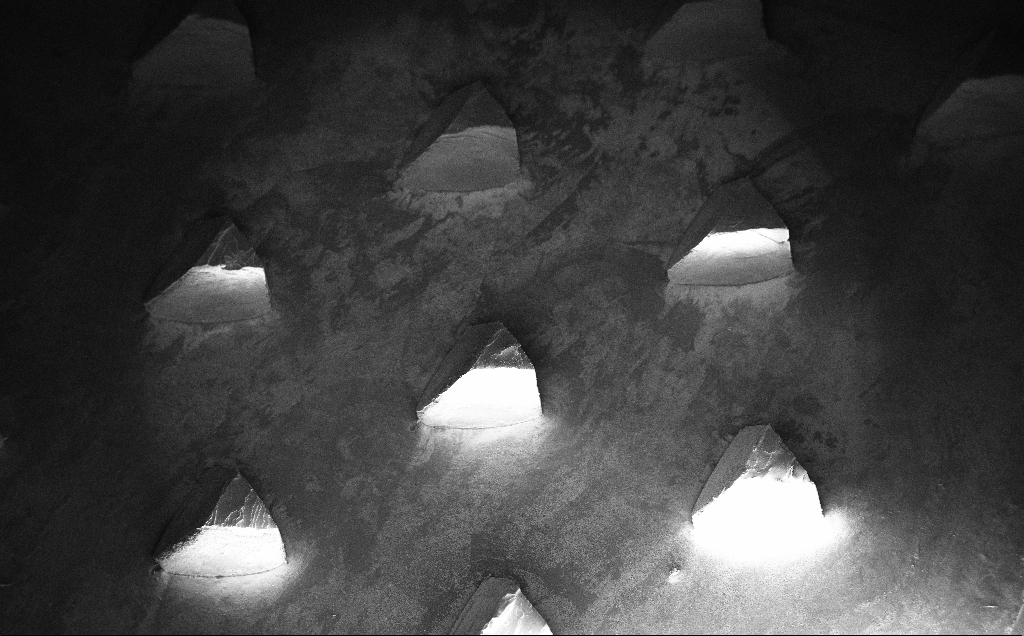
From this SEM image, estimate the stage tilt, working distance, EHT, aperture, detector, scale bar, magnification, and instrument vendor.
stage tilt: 30°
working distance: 9 mm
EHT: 10 kV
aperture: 30 µm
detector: InLens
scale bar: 200000 nm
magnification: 0.08 K X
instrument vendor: Zeiss